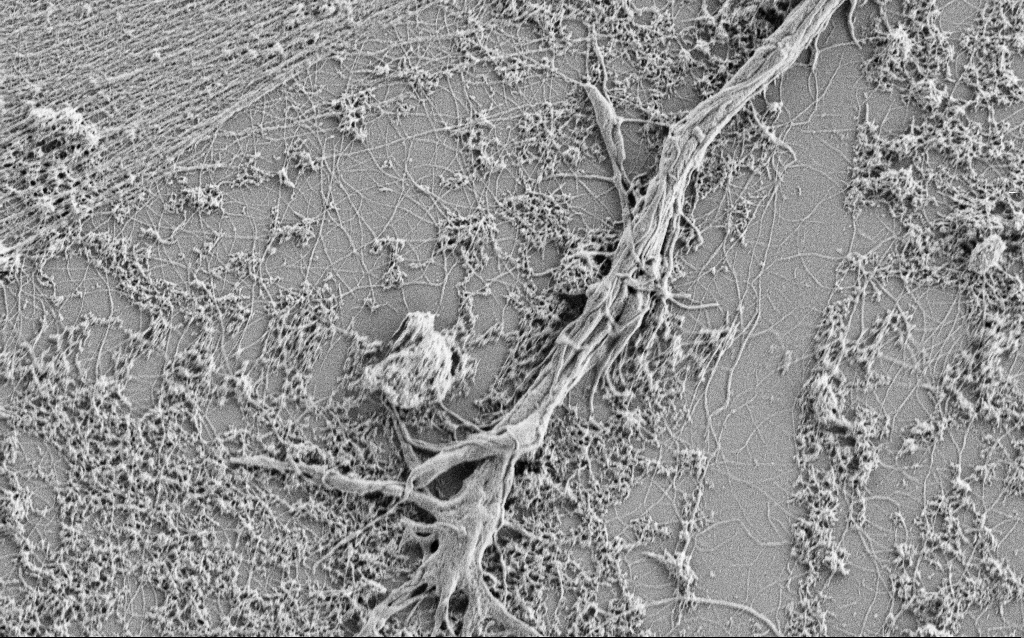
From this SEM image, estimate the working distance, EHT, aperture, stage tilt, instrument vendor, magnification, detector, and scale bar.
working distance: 4 mm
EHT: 1 kV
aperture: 30 µm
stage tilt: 0°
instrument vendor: Zeiss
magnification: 10 K X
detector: SE2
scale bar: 2000 nm